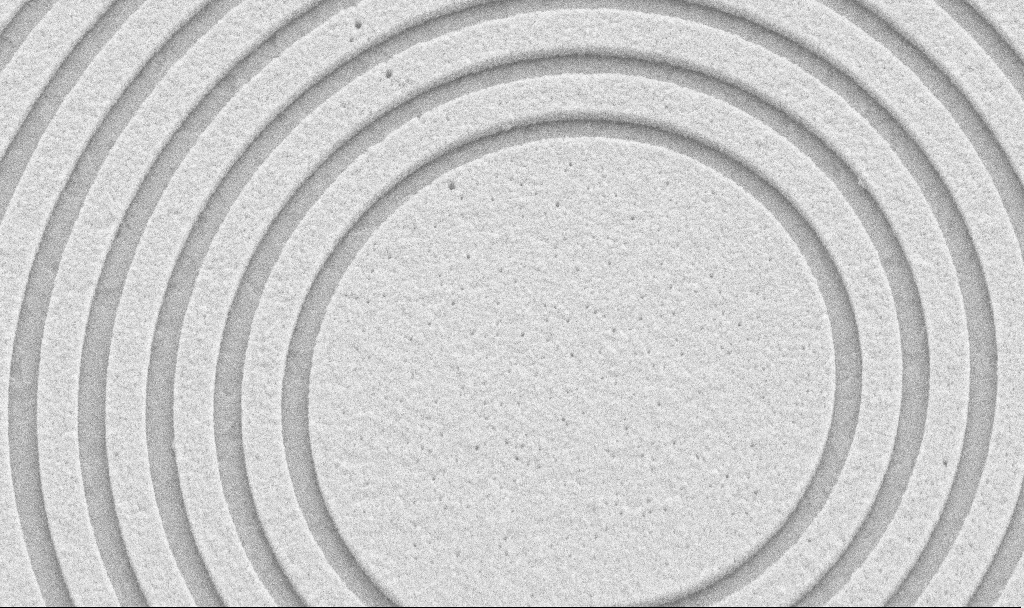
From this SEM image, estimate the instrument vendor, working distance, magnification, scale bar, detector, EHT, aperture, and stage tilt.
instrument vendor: Zeiss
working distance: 9.5 mm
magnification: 38.7 K X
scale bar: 1000 nm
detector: SE2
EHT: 5 kV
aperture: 30 µm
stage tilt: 45°